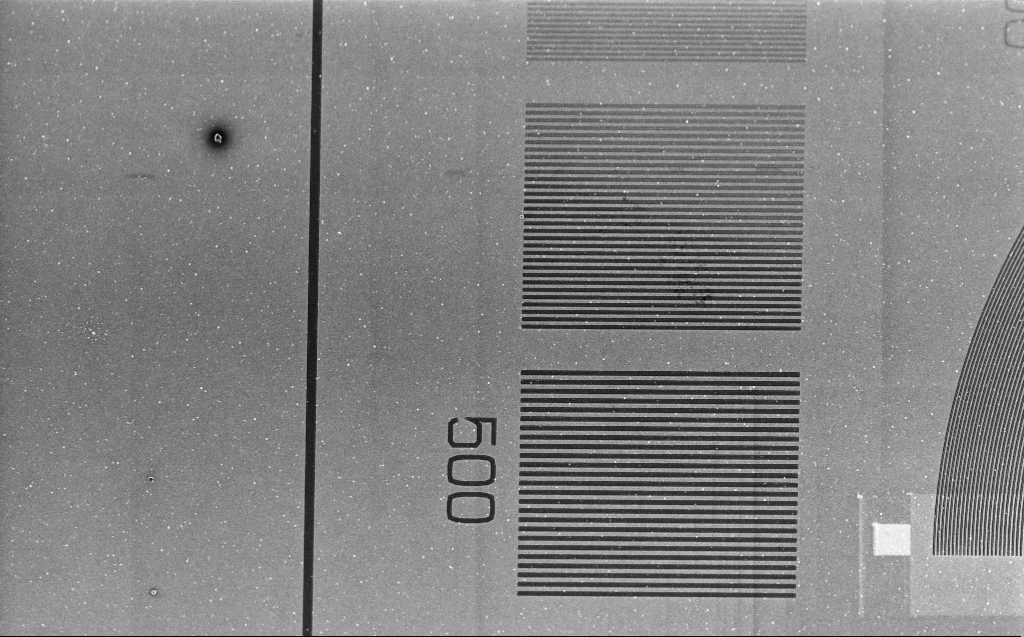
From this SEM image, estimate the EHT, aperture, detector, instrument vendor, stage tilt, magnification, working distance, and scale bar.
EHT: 1 kV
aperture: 30 µm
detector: InLens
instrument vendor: Zeiss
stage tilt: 30°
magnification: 3.46 K X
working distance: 4 mm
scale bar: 10000 nm